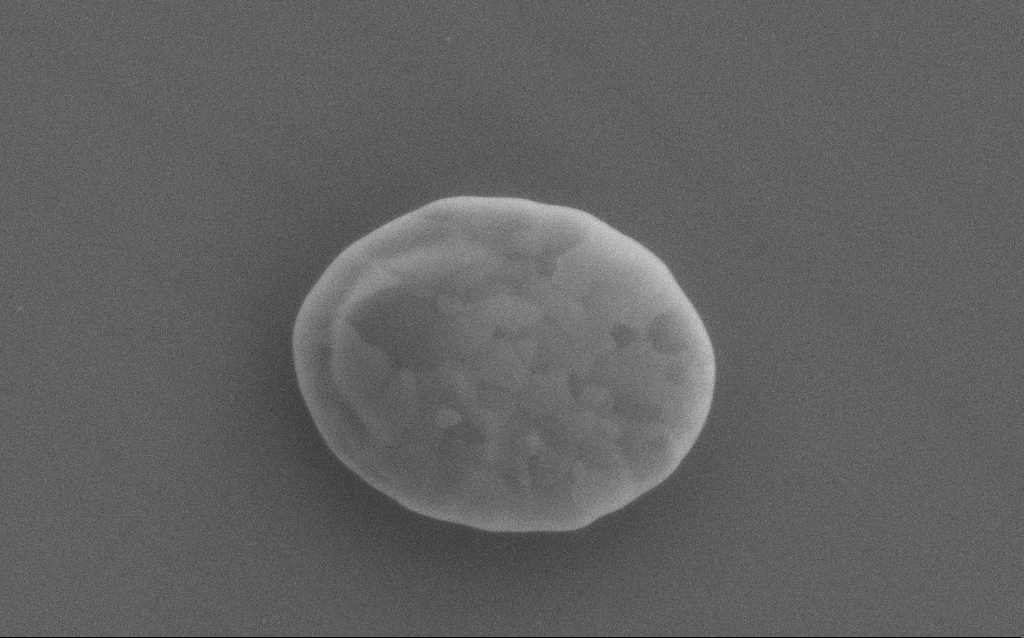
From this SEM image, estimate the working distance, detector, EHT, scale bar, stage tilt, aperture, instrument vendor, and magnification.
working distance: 3 mm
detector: SE2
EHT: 5 kV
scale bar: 1000 nm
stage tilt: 0°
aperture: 30 µm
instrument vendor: Zeiss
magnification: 52.76 K X